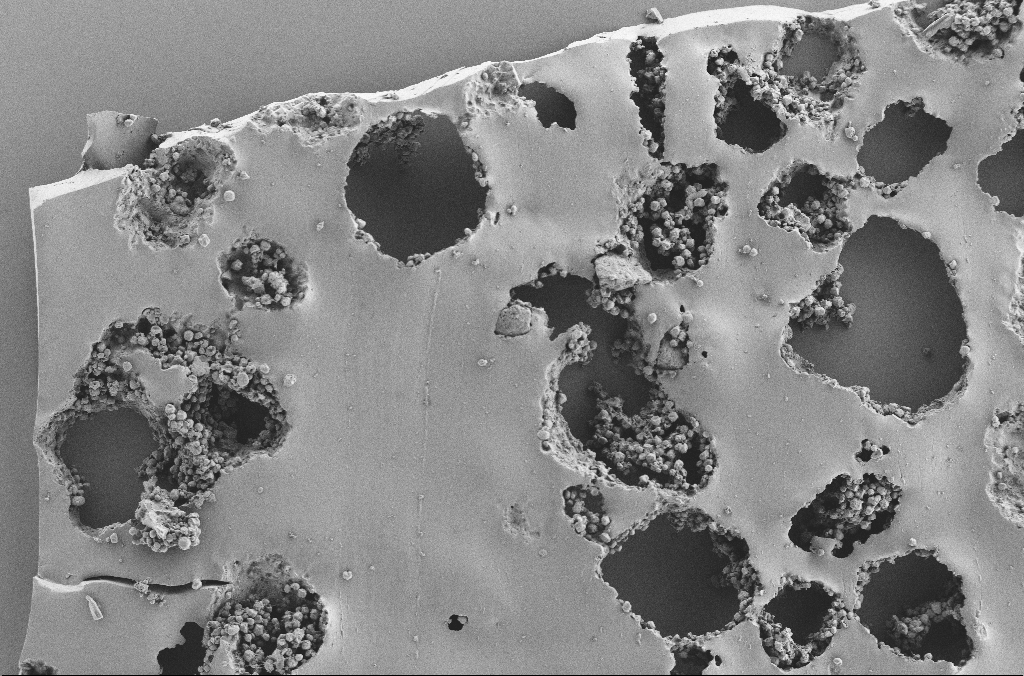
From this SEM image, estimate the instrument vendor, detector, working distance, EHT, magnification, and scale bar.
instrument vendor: Zeiss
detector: SE2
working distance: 3.7 mm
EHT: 2 kV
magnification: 0.25 K X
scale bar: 100000 nm